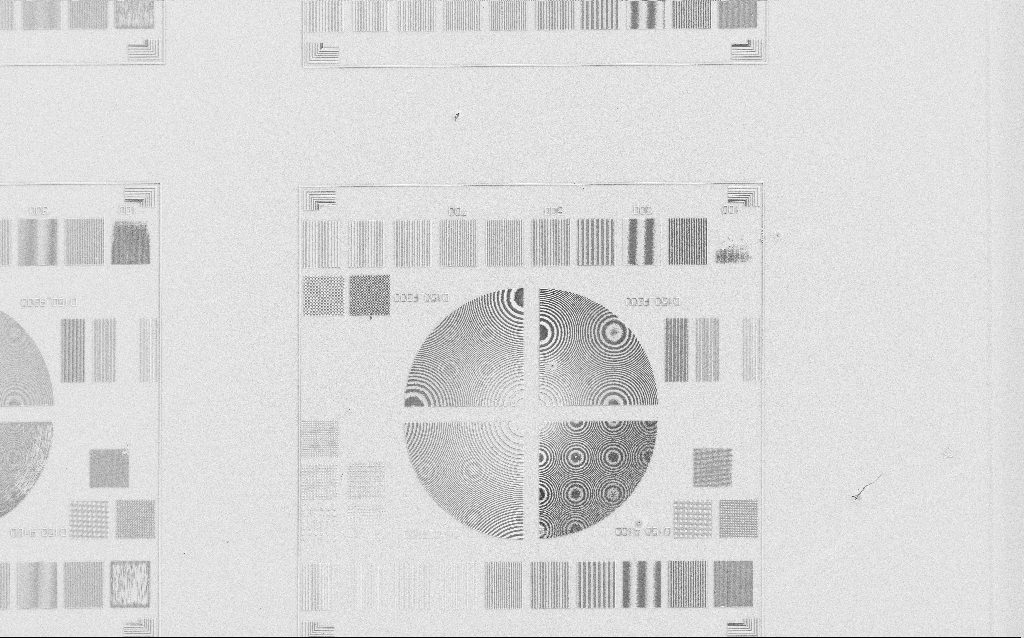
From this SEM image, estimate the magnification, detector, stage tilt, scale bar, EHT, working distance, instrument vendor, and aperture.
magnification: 0.58 K X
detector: SE2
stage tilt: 0°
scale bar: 100000 nm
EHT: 3 kV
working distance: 8 mm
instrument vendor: Zeiss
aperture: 30 µm